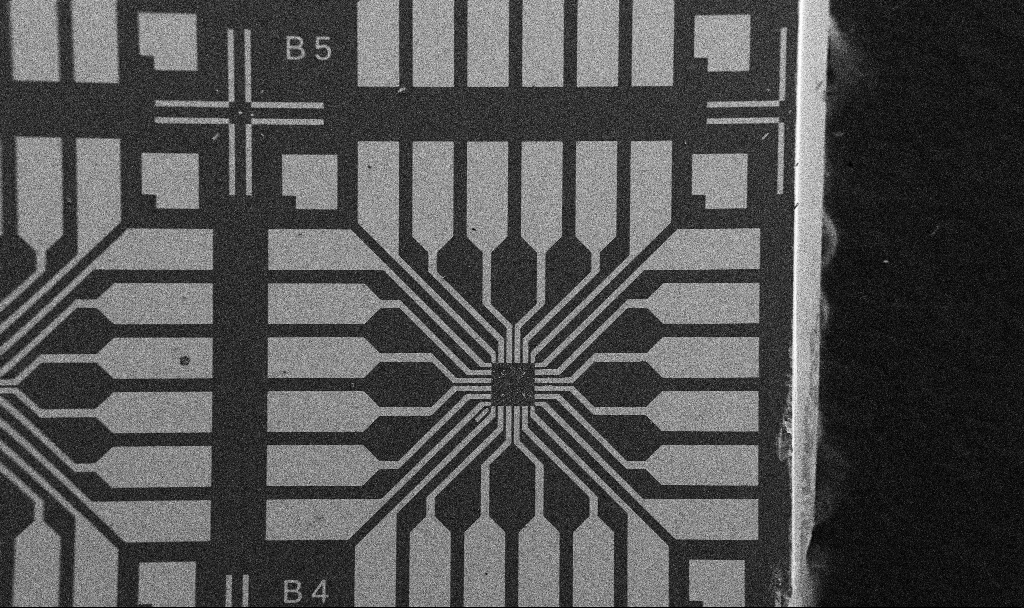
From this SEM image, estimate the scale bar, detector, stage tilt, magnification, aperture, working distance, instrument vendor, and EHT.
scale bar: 200000 nm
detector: SE2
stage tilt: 0°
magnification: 0.1 K X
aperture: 30 µm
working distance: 10.7 mm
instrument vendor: Zeiss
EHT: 5 kV